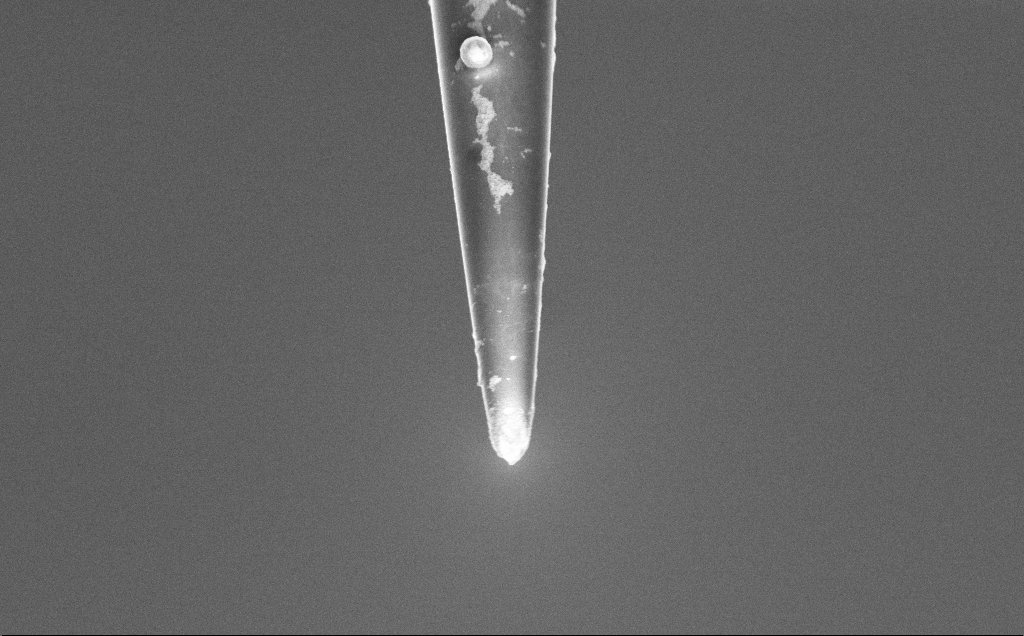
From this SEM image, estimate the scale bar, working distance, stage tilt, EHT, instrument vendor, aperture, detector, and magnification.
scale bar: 1000 nm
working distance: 7.5 mm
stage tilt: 45°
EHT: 3 kV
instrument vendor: Zeiss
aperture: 30 µm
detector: InLens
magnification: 25 K X